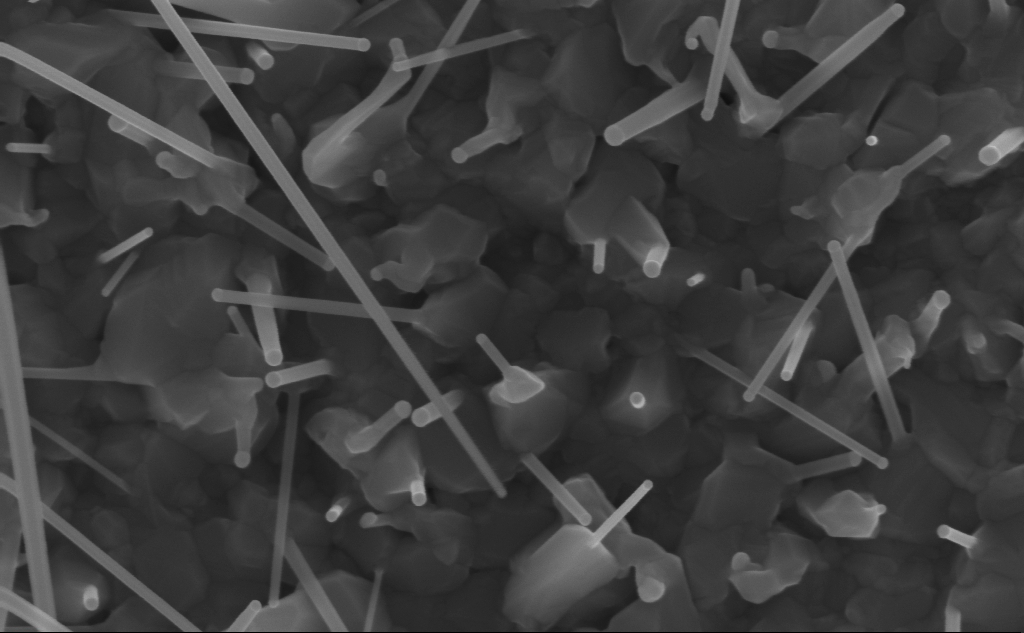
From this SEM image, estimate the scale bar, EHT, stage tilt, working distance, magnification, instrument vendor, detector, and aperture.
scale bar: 200 nm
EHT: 10 kV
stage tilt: -0.2°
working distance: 7 mm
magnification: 102.67 K X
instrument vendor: Zeiss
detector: InLens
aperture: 30 µm